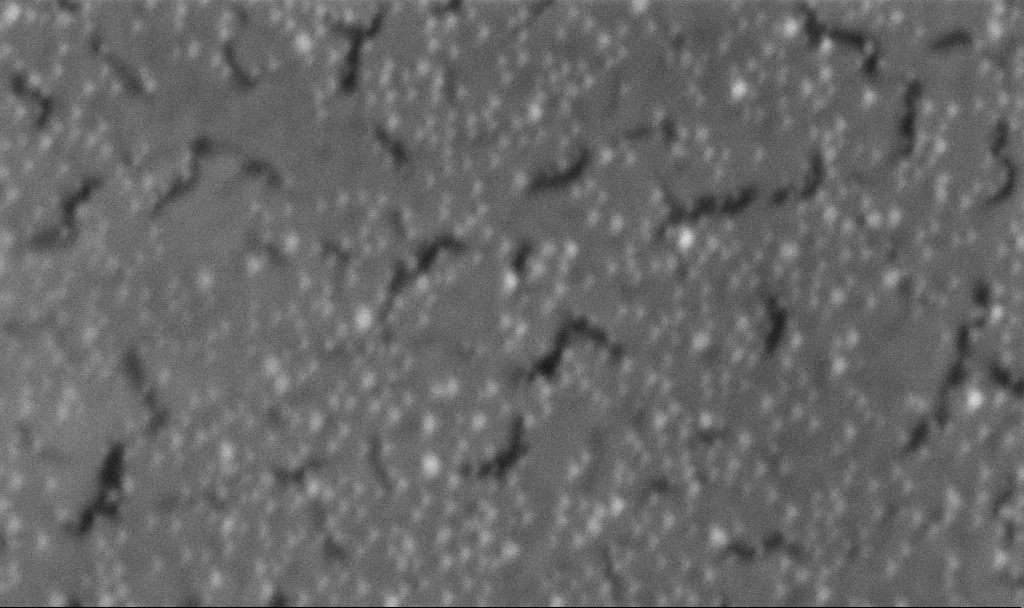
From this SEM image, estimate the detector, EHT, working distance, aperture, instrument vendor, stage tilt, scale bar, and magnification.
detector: InLens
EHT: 5 kV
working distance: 3.3 mm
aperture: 30 µm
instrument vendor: Zeiss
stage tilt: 0°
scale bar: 200 nm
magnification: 242.51 K X